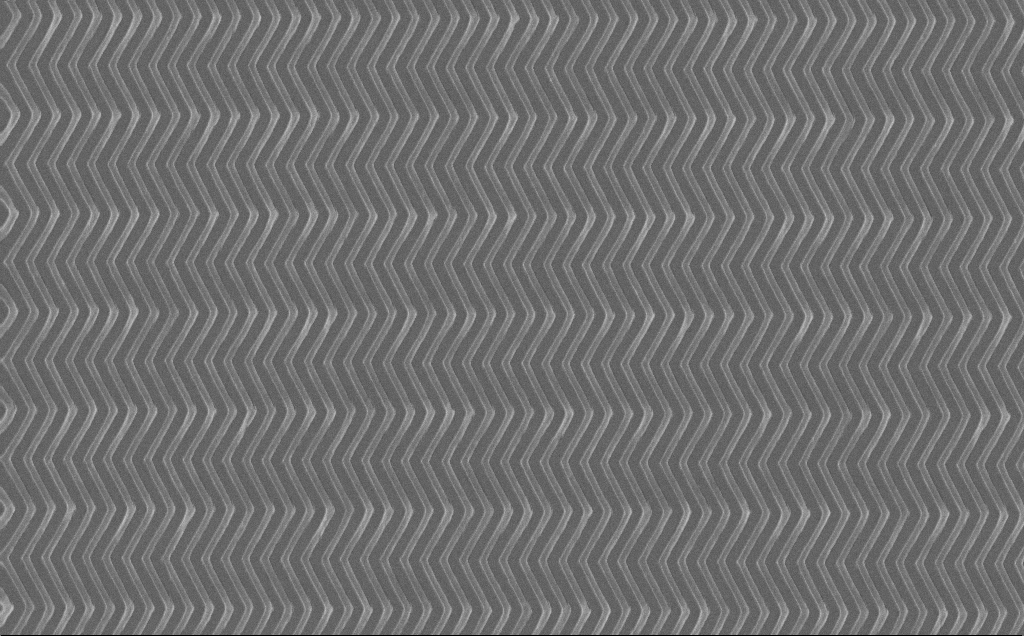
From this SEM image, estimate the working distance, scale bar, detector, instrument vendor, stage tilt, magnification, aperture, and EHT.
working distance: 7 mm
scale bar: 2000 nm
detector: InLens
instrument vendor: Zeiss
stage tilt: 0°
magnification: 18.26 K X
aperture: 30 µm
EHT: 10 kV